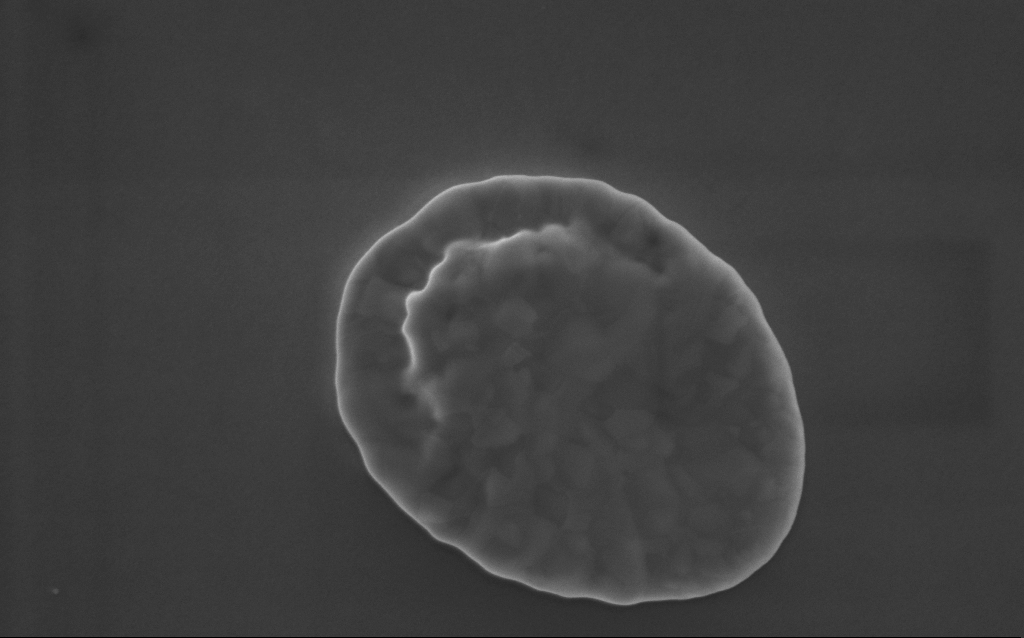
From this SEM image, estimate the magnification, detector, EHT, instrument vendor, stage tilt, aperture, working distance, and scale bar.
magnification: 90 K X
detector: InLens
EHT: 5 kV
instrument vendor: Zeiss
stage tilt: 0°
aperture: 30 µm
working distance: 3 mm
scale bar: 200 nm